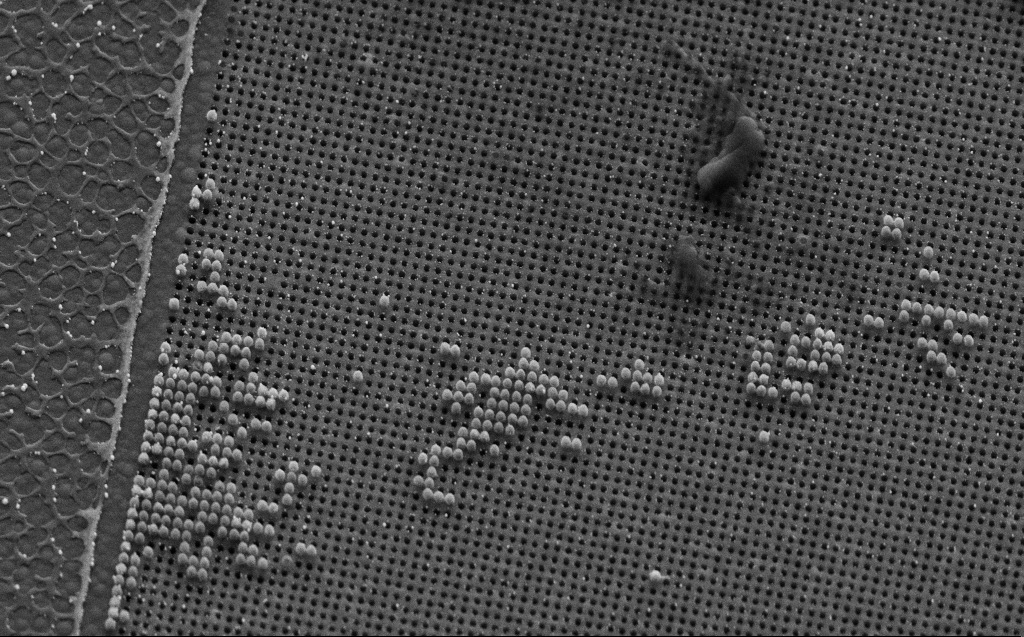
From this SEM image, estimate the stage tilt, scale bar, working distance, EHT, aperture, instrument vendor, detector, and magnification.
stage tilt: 45°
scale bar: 2000 nm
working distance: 9 mm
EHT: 5 kV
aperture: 30 µm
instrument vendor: Zeiss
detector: SE2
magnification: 20.88 K X